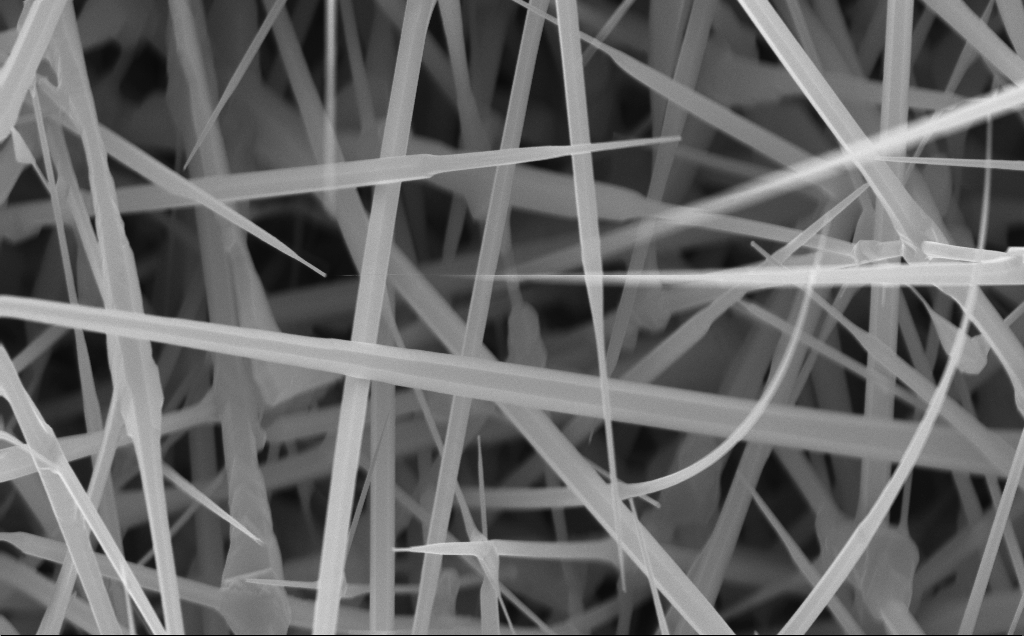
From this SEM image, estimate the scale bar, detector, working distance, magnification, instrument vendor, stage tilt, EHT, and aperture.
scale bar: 1000 nm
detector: InLens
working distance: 4 mm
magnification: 40 K X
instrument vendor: Zeiss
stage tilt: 0°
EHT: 10 kV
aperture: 30 µm